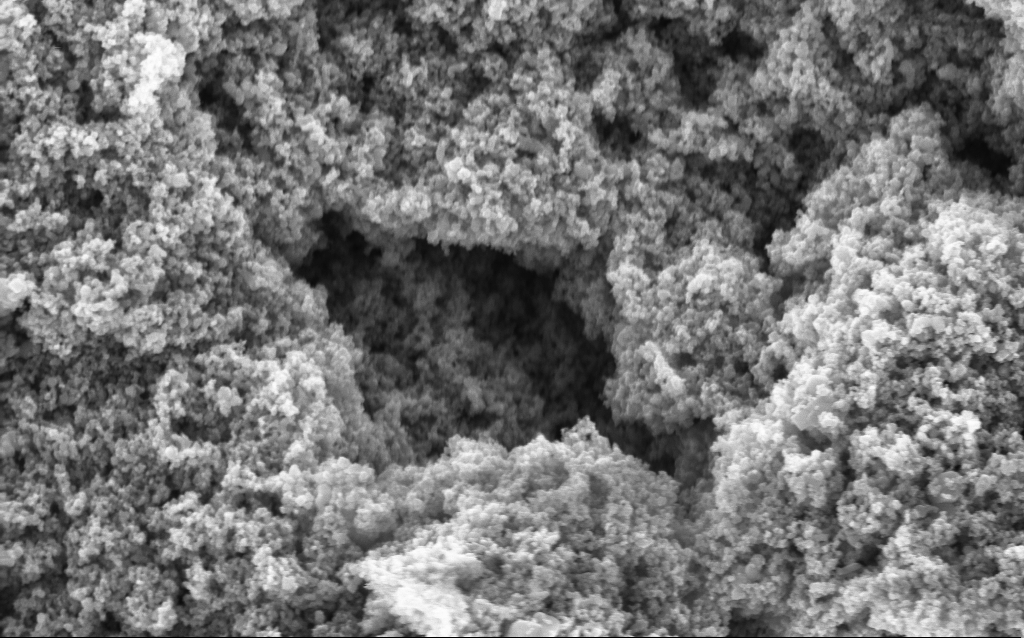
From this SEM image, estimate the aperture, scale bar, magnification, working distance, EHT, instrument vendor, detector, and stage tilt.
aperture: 30 µm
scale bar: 1000 nm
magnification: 68.69 K X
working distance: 4.4 mm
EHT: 5 kV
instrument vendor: Zeiss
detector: InLens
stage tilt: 0°